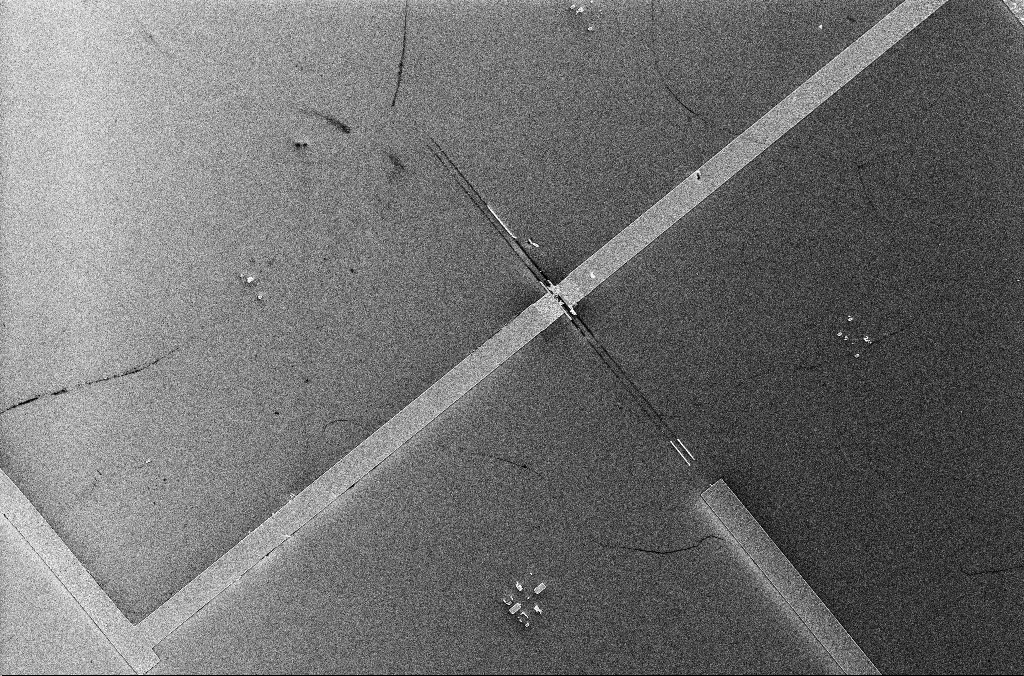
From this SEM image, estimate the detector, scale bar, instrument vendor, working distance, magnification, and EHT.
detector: InLens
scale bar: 20000 nm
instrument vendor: Zeiss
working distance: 3.3 mm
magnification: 1.97 K X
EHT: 5 kV